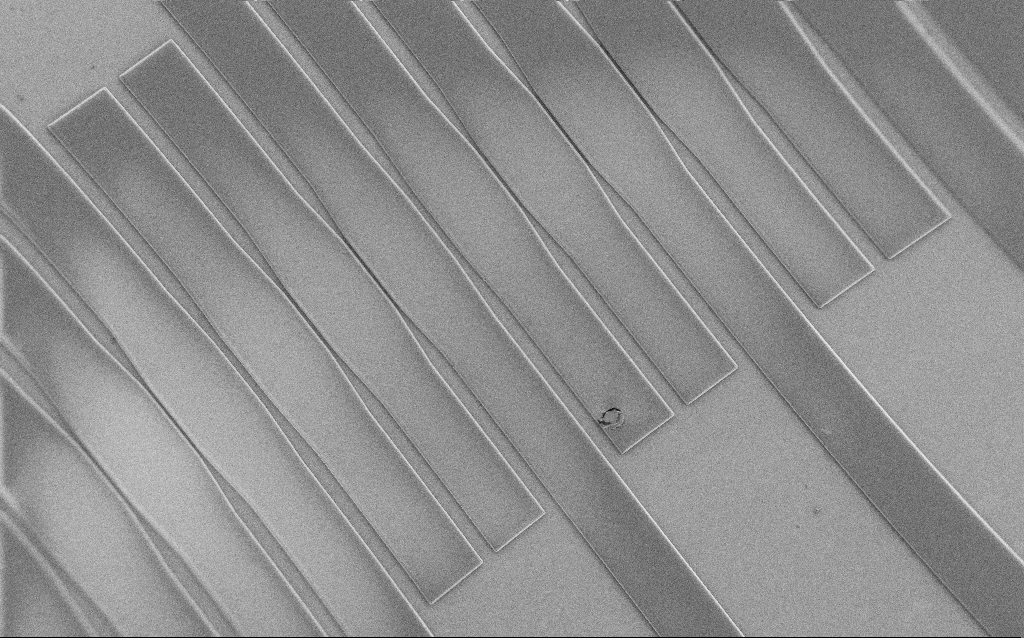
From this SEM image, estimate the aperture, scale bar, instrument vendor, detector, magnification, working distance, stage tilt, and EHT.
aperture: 30 µm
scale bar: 200000 nm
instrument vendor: Zeiss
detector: SE2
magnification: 0.276 K X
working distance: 7 mm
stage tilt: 0°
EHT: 1 kV